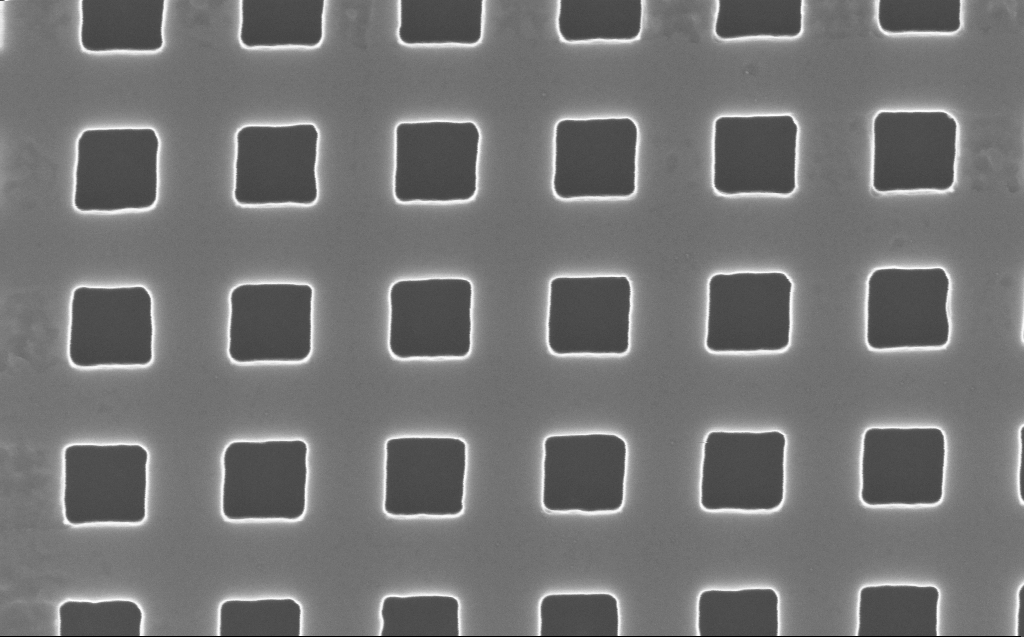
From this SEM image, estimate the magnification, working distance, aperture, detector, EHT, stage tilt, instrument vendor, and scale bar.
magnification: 118.54 K X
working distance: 4 mm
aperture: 30 µm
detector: InLens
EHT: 10 kV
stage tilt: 0°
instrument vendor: Zeiss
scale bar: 200 nm